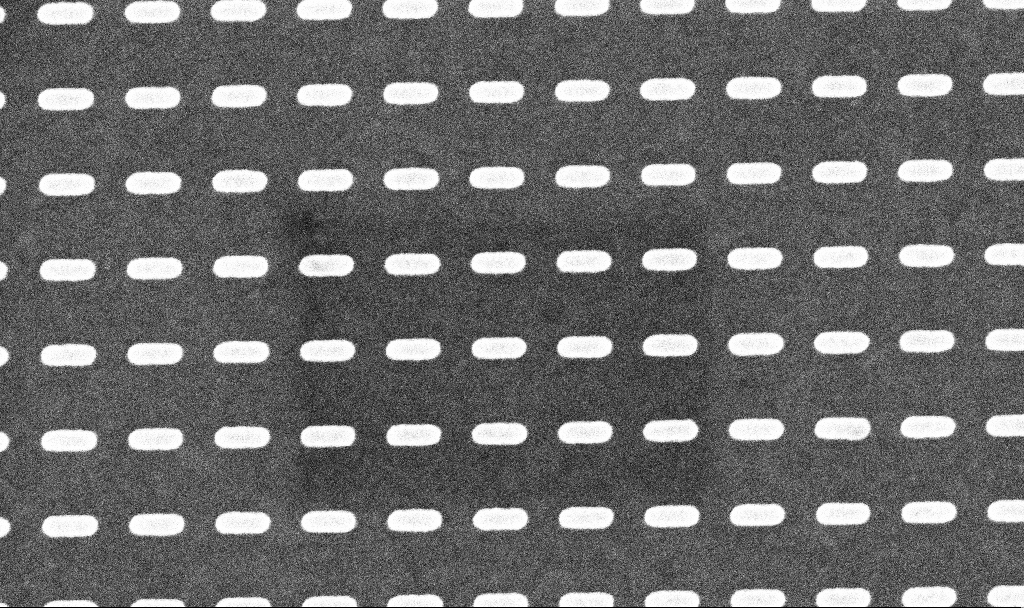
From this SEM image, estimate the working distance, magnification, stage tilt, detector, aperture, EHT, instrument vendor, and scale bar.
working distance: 4.6 mm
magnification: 105.21 K X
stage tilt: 0°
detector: InLens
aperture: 30 µm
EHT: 5 kV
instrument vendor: Zeiss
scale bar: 200 nm